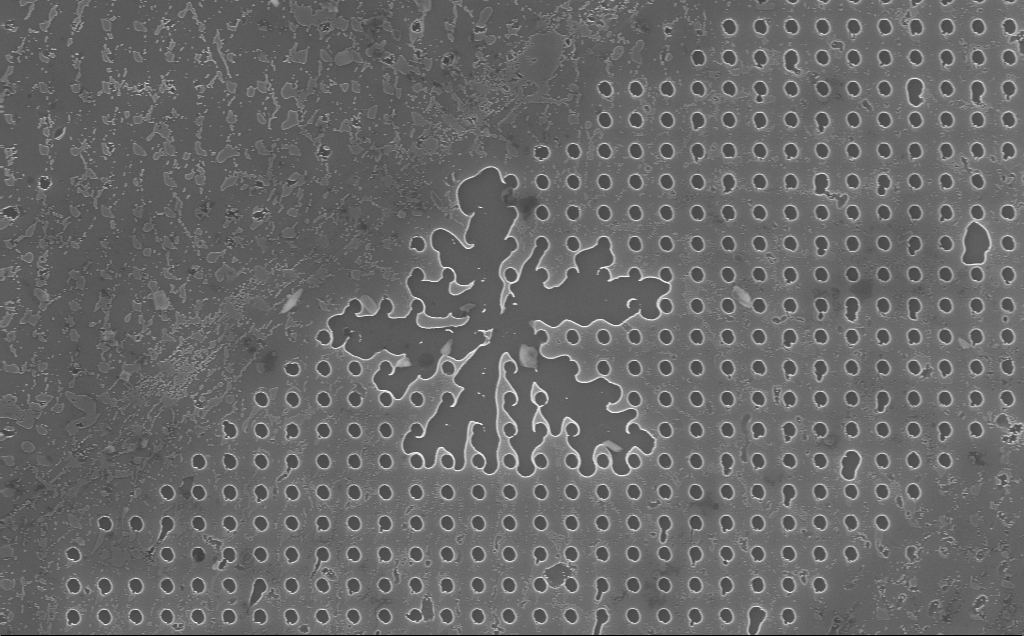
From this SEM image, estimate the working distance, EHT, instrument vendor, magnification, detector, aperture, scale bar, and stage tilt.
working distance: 4 mm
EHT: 10 kV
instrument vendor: Zeiss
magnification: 10.94 K X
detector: InLens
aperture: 30 µm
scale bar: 2000 nm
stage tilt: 0°